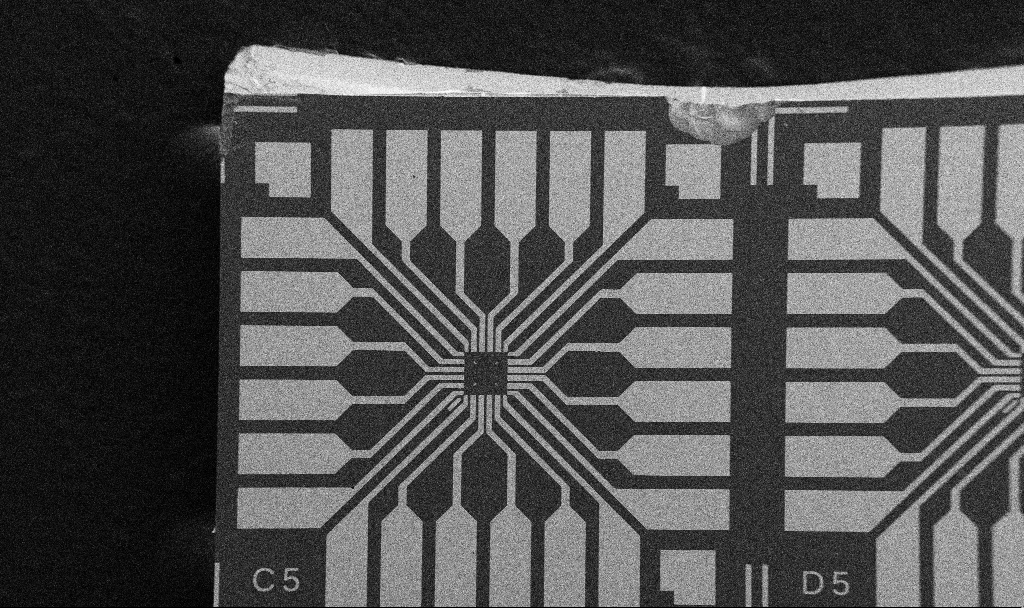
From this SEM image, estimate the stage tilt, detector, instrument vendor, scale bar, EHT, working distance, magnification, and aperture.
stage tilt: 0°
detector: SE2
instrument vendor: Zeiss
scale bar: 200000 nm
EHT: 5 kV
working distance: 10.7 mm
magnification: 0.1 K X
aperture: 30 µm